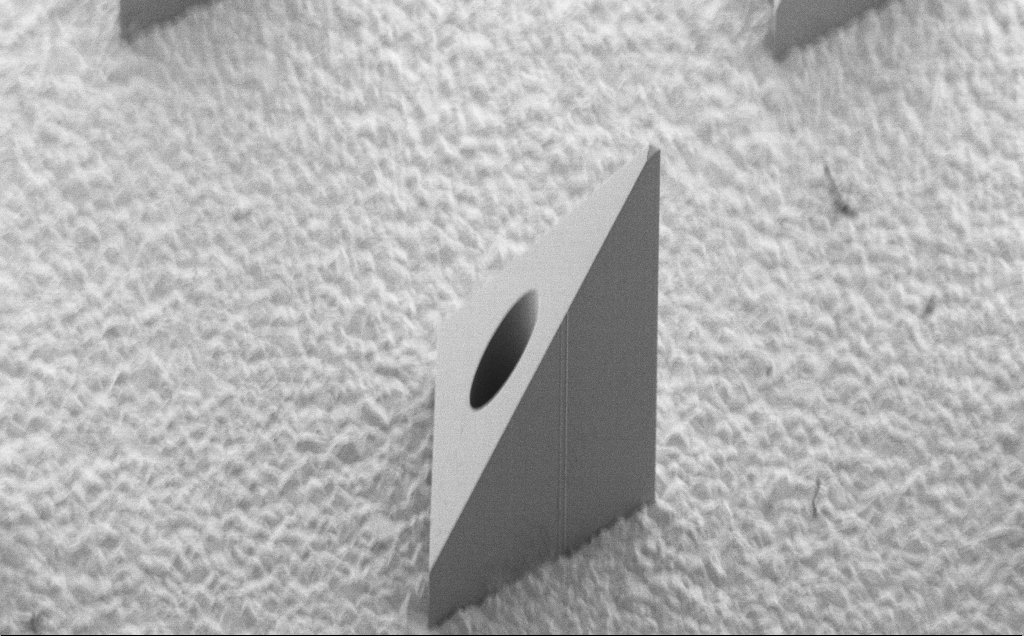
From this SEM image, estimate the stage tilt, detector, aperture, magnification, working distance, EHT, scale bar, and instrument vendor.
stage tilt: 40°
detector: SE2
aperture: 30 µm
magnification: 0.16 K X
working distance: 8 mm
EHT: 5 kV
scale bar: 200000 nm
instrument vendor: Zeiss